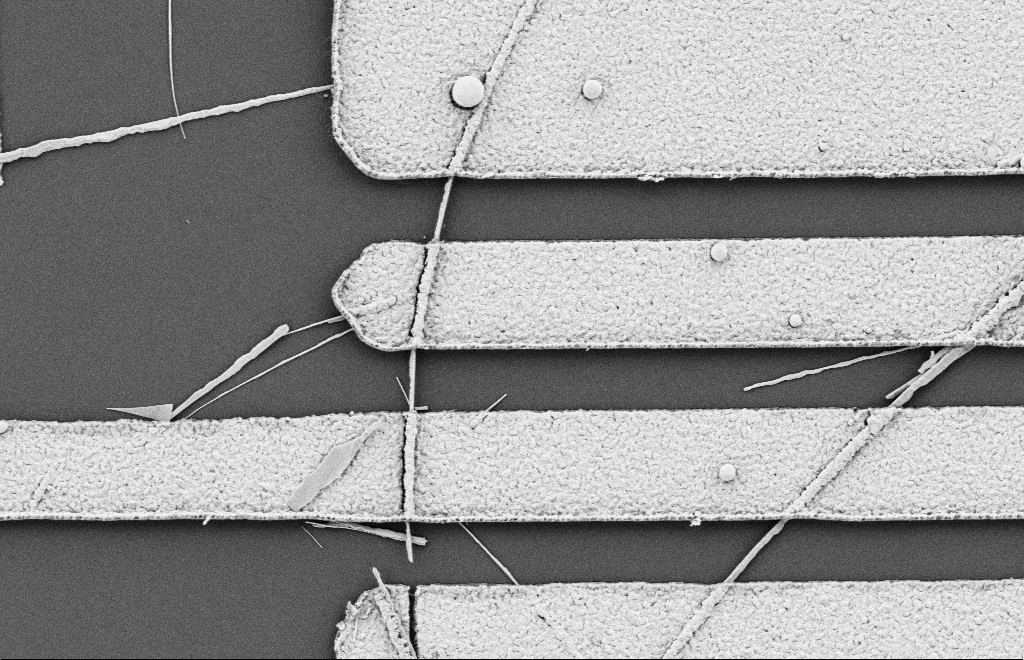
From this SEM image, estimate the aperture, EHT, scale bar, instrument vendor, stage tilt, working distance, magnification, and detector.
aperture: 20 µm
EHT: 2 kV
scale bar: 2000 nm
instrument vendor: Zeiss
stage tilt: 0°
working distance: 10 mm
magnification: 15.64 K X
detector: SE2